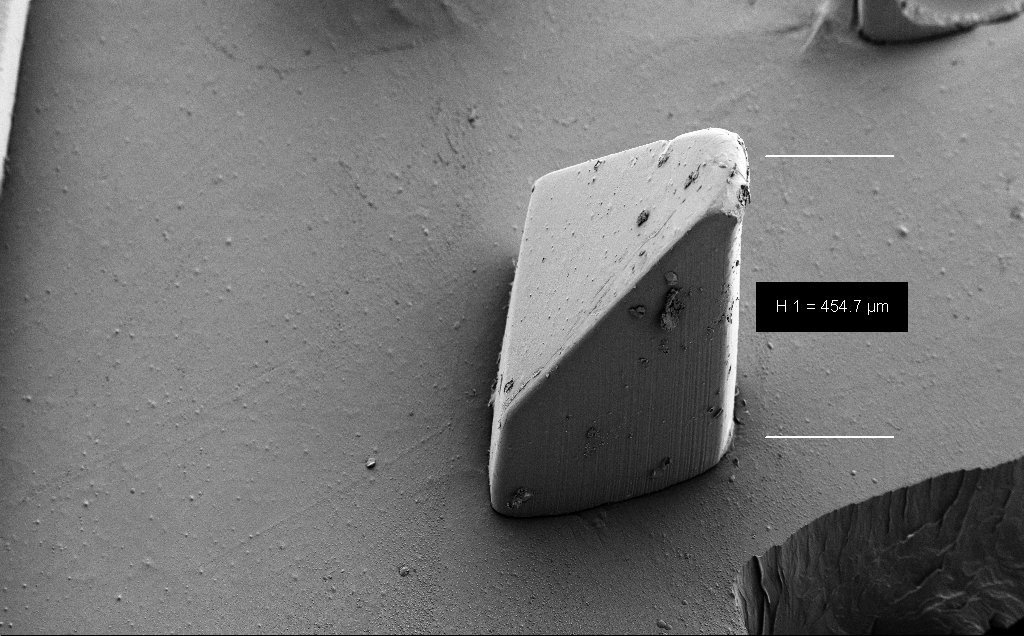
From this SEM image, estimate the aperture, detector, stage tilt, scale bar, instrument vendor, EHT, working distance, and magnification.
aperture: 30 µm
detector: SE2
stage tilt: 40°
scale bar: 100000 nm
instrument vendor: Zeiss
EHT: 5 kV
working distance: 9 mm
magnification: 0.227 K X